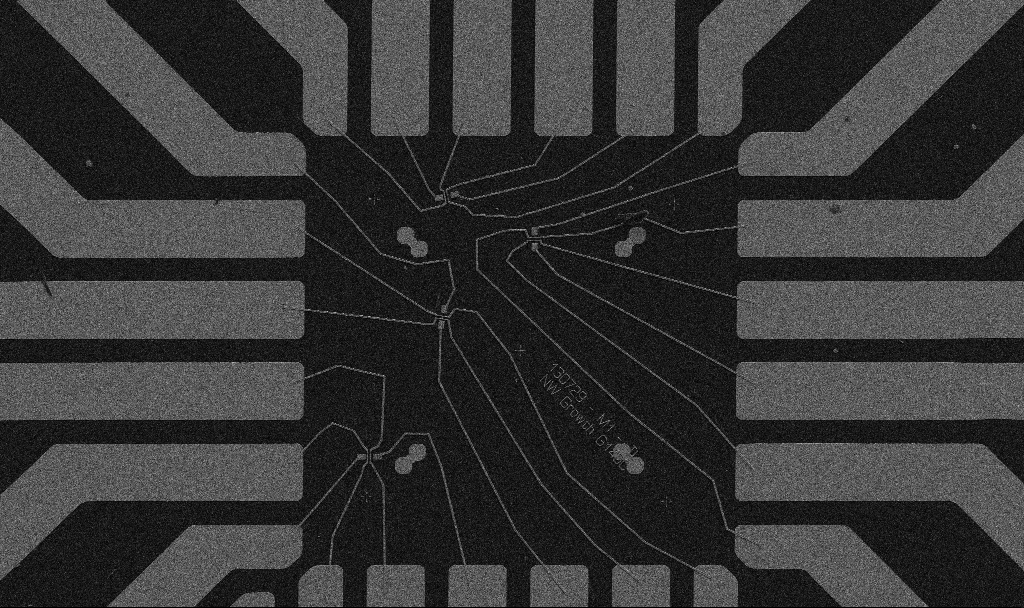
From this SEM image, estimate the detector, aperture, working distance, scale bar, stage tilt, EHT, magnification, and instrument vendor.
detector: SE2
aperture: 30 µm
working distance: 9.7 mm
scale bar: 20000 nm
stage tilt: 0°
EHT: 5 kV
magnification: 1 K X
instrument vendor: Zeiss